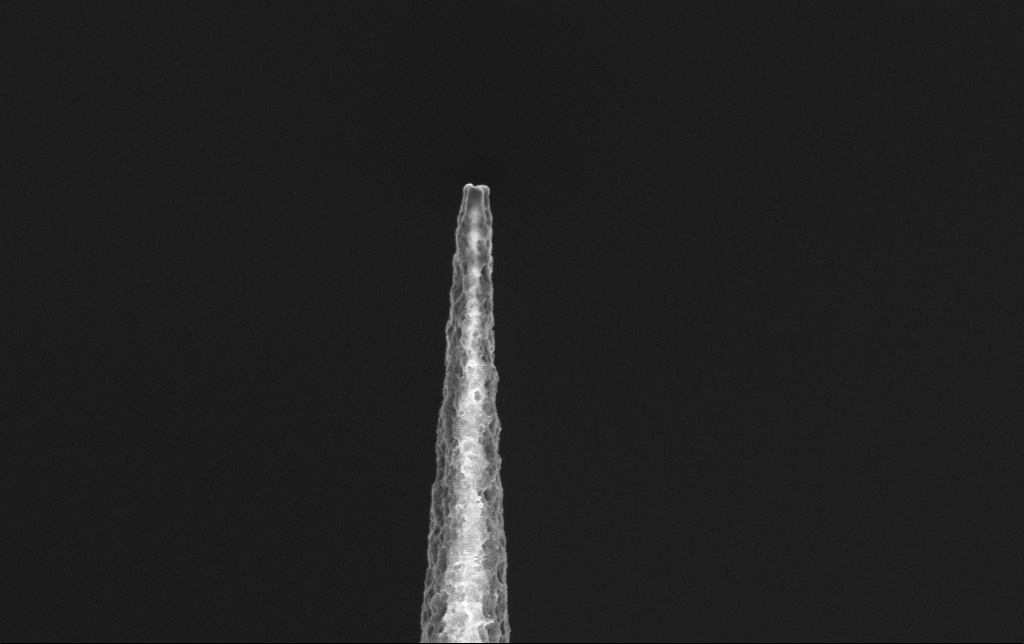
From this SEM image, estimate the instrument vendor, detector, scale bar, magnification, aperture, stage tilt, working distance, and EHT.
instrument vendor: Zeiss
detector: InLens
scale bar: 1000 nm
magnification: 50 K X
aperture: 30 µm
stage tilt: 0°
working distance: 6.5 mm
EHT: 2 kV